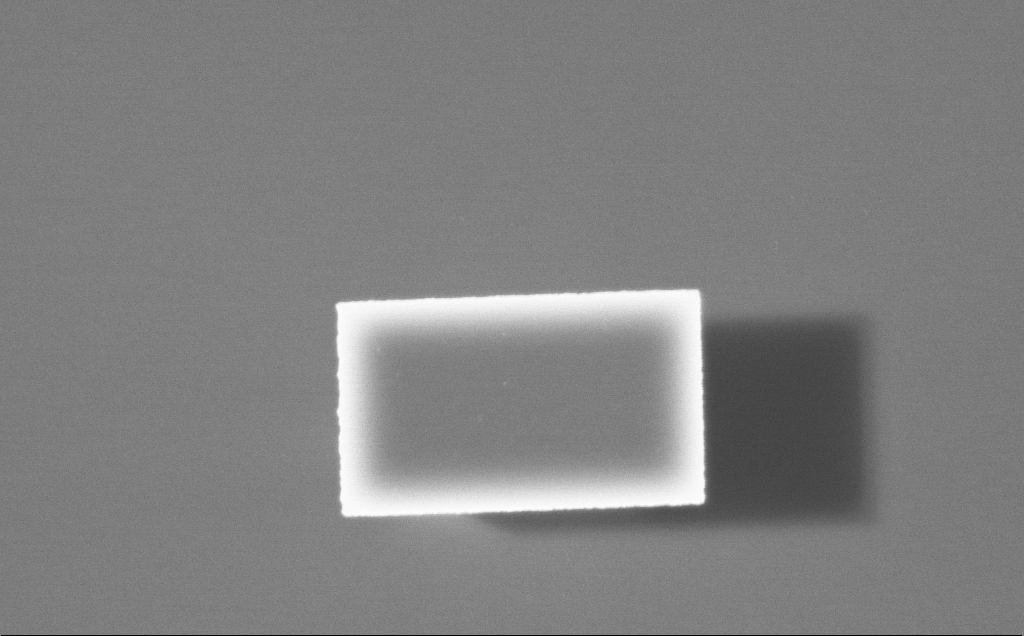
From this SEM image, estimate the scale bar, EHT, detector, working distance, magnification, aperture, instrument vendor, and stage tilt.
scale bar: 2000 nm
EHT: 7.5 kV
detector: InLens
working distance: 4 mm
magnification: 27.73 K X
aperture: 30 µm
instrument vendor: Zeiss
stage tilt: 0°